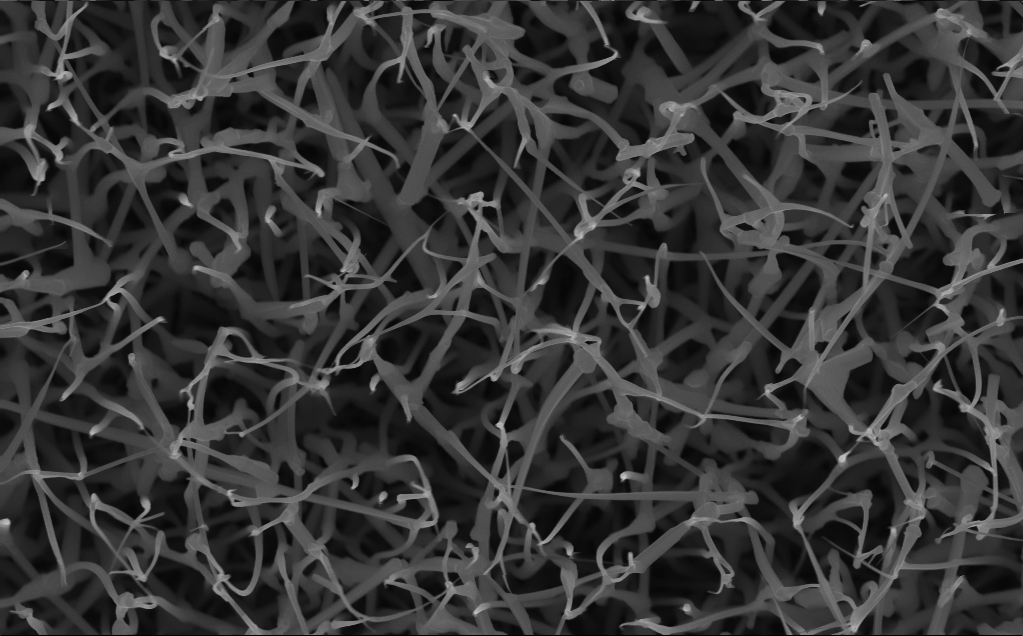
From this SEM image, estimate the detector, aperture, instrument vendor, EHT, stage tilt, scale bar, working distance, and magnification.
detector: InLens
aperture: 30 µm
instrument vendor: Zeiss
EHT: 10 kV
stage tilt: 0°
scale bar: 1000 nm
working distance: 5 mm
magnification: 40 K X